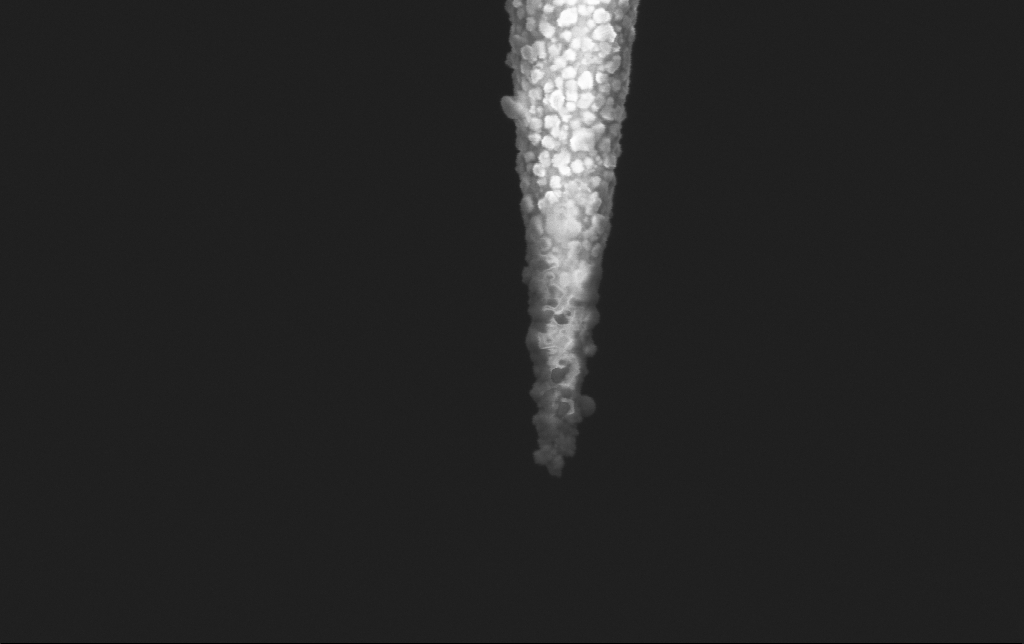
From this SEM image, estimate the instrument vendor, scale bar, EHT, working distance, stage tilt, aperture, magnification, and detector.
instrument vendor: Zeiss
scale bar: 200 nm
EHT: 2 kV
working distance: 5.9 mm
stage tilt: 0°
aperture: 30 µm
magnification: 100 K X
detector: InLens